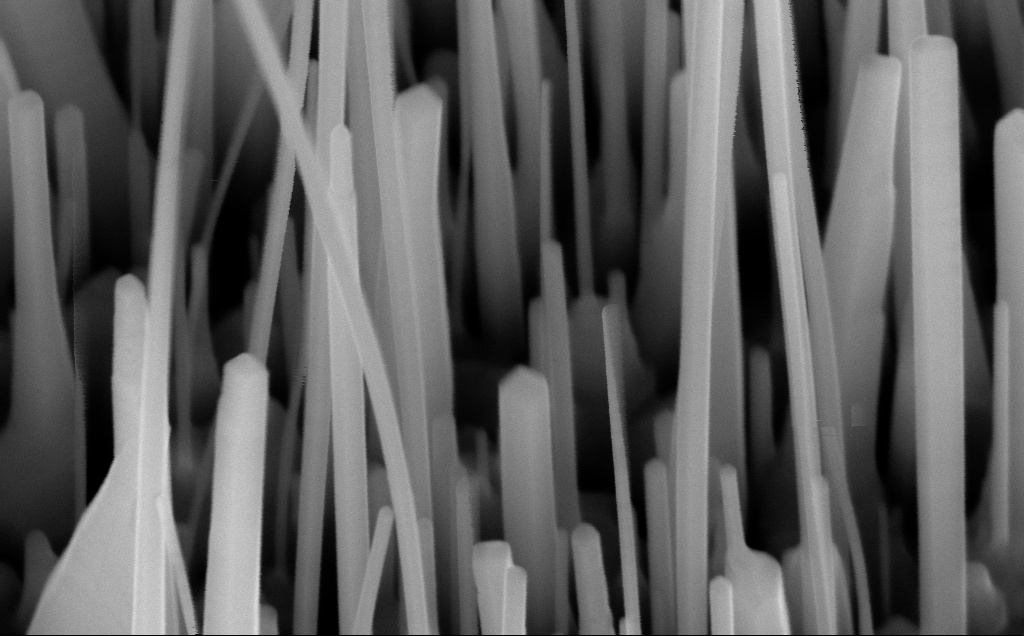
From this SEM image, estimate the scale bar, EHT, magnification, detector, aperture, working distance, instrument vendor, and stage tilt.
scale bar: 100 nm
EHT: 10 kV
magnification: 200 K X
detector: InLens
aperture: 30 µm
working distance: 4 mm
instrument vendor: Zeiss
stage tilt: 45°